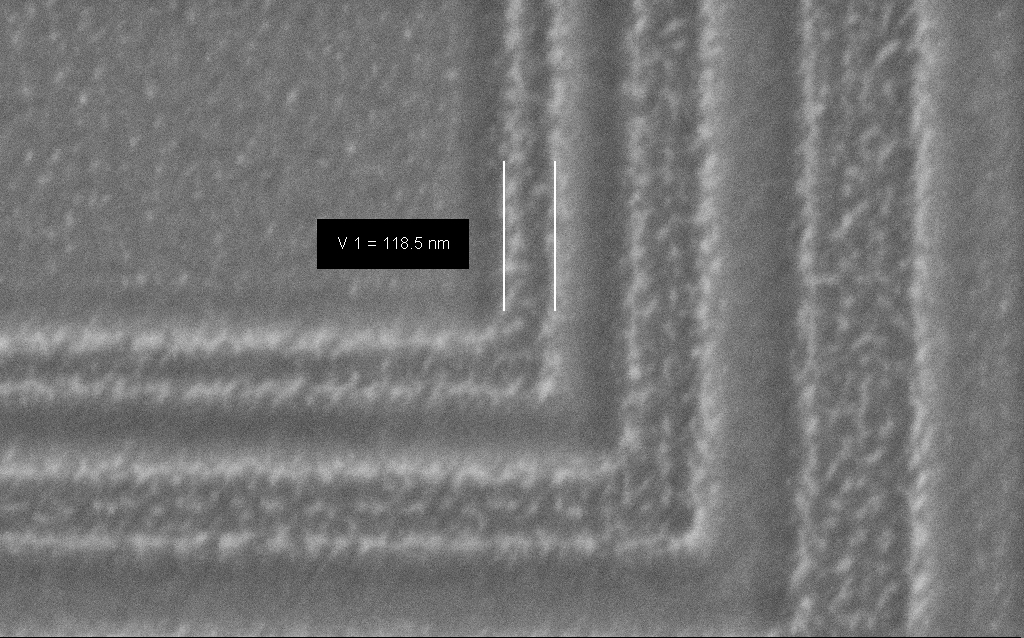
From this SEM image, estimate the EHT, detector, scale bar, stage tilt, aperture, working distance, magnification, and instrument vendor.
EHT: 1.5 kV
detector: SE2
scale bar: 200 nm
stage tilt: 0°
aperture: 30 µm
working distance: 6 mm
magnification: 157.98 K X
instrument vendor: Zeiss